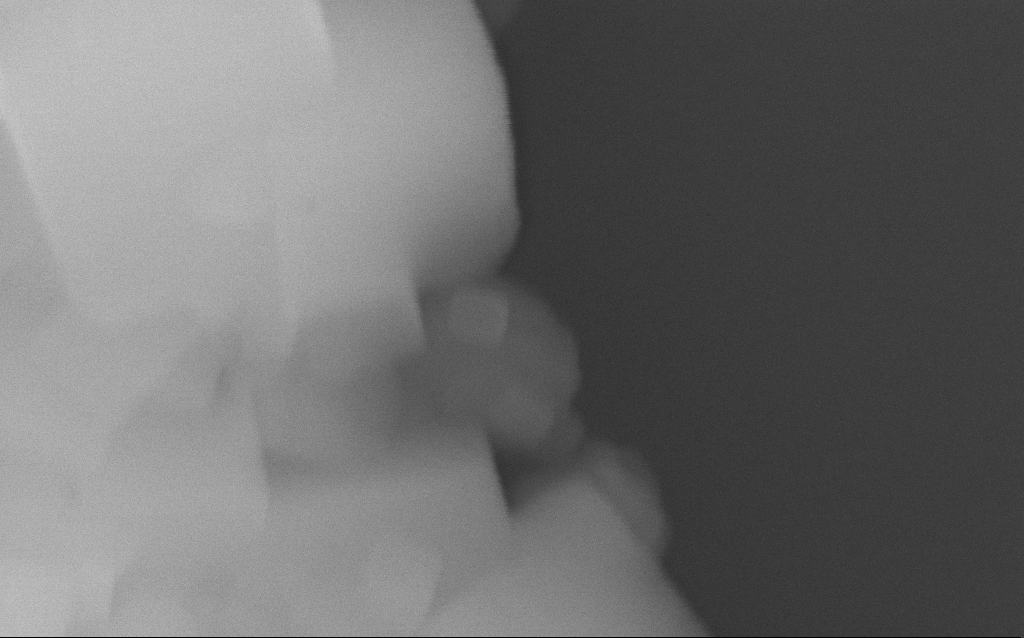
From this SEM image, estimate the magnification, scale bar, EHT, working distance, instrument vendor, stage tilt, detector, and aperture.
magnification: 307.3 K X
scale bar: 200 nm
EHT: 10 kV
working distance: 3 mm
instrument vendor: Zeiss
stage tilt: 0°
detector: InLens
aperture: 30 µm